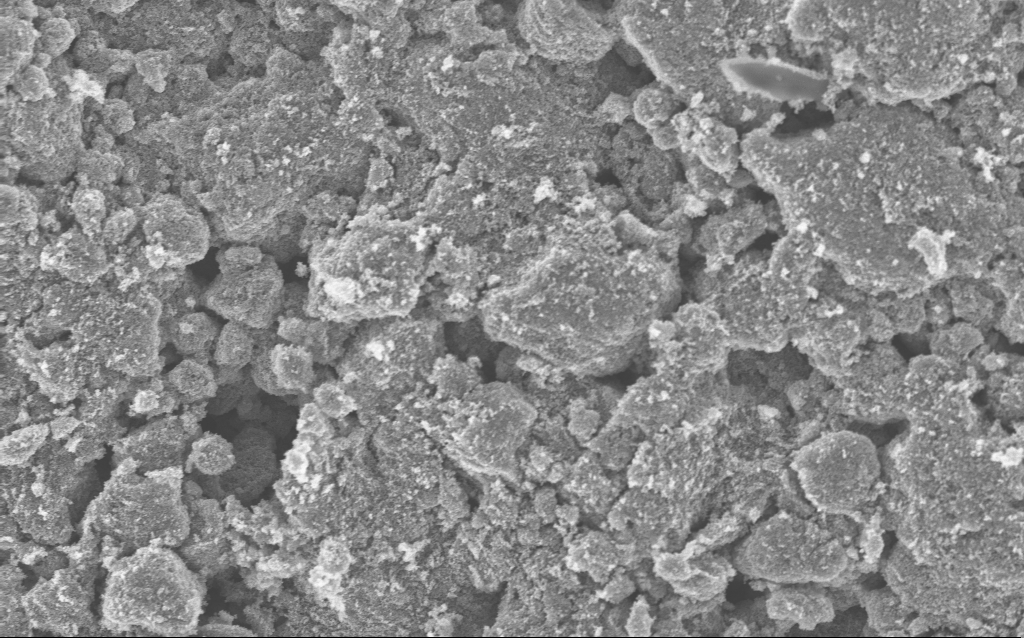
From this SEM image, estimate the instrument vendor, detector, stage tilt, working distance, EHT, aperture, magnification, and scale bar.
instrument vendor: Zeiss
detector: InLens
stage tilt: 0°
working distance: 4.7 mm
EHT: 5 kV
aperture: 30 µm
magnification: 12.08 K X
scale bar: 1000 nm